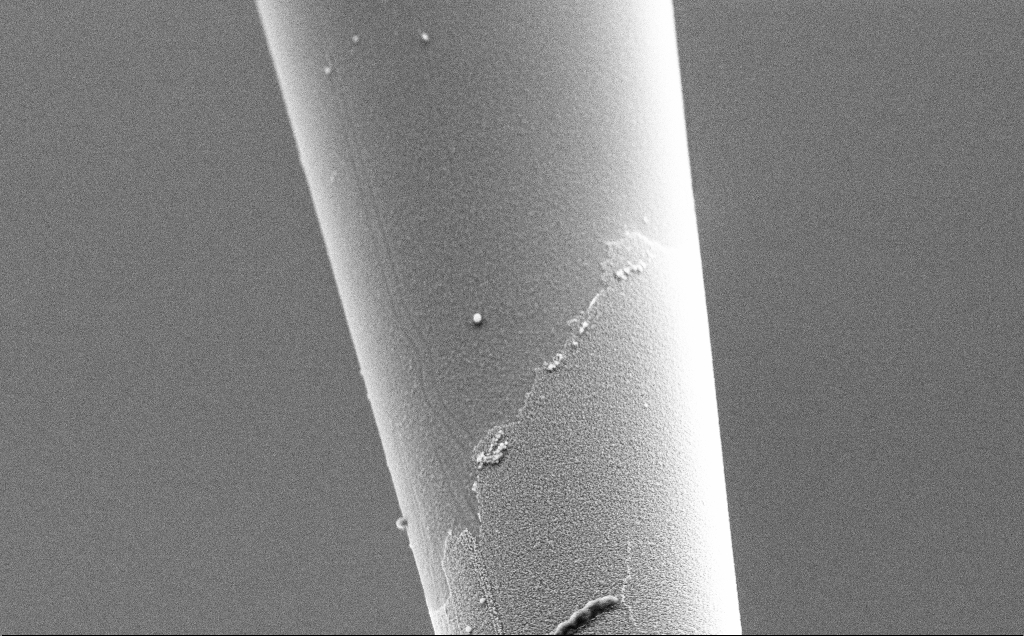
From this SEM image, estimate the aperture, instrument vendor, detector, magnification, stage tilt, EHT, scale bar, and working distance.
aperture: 30 µm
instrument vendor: Zeiss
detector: SE2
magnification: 15 K X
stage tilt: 45°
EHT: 3 kV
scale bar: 1000 nm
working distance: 7.8 mm